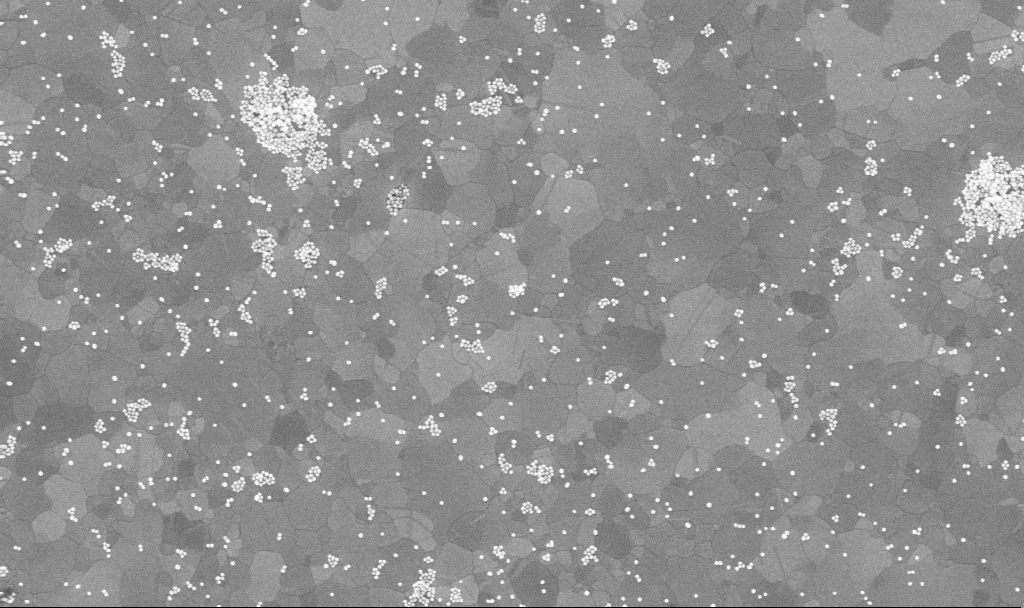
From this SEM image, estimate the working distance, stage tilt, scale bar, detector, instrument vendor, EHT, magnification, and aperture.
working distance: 3.8 mm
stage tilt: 0°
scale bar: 200 nm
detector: InLens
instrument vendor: Zeiss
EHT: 10 kV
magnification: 80 K X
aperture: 30 µm